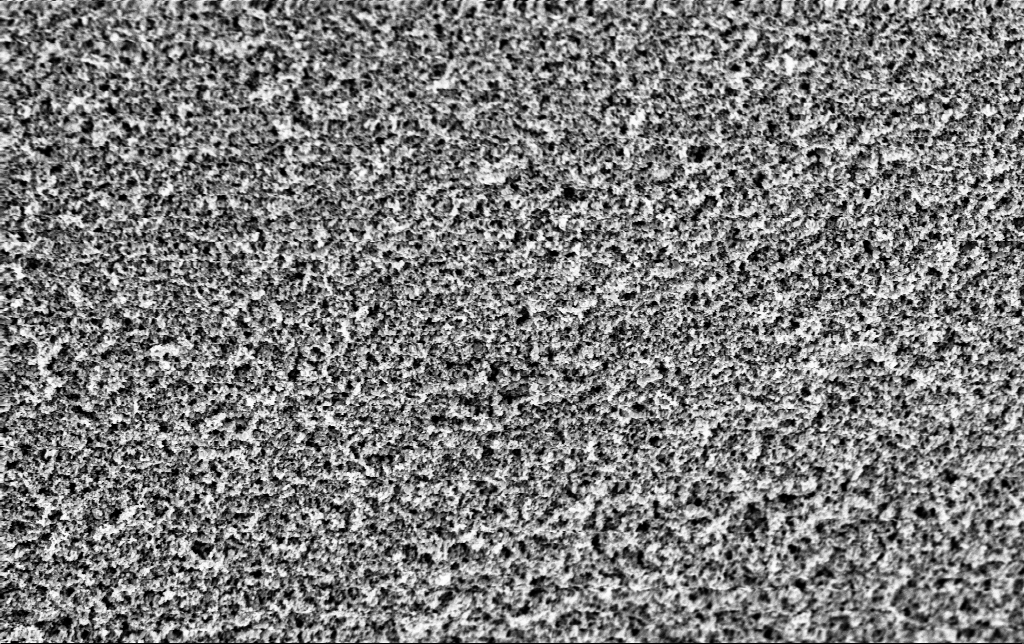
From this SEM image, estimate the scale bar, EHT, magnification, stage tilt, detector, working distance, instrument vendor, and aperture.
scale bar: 2000 nm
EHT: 3 kV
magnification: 30 K X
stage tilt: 0°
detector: InLens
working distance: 2.8 mm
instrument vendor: Zeiss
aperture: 30 µm